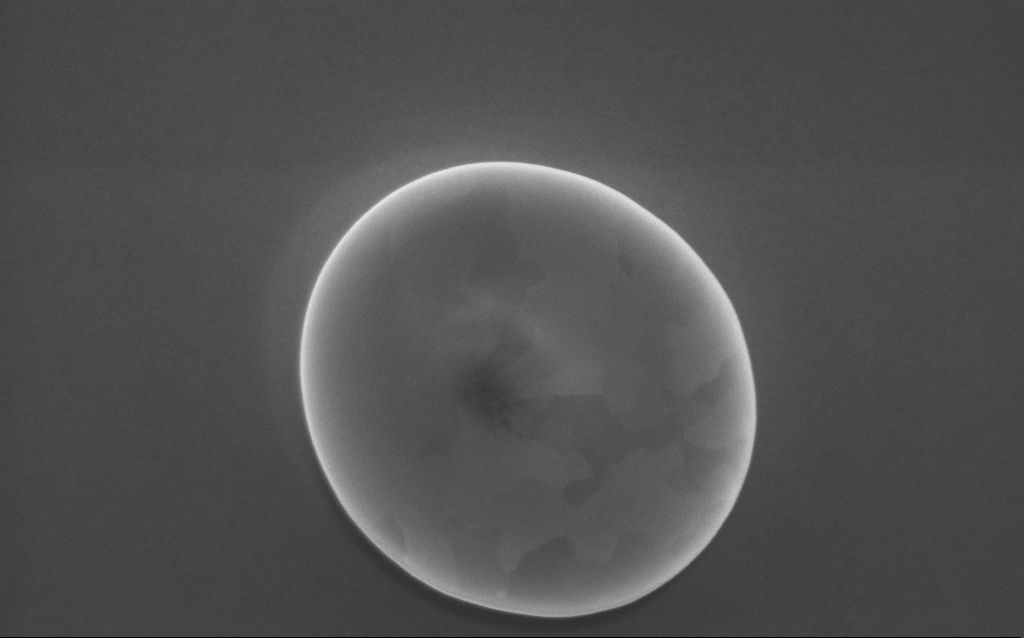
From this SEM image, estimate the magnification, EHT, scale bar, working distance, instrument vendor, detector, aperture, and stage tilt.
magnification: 100 K X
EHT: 5 kV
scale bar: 200 nm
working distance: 3 mm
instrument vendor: Zeiss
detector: InLens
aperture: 30 µm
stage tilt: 0°